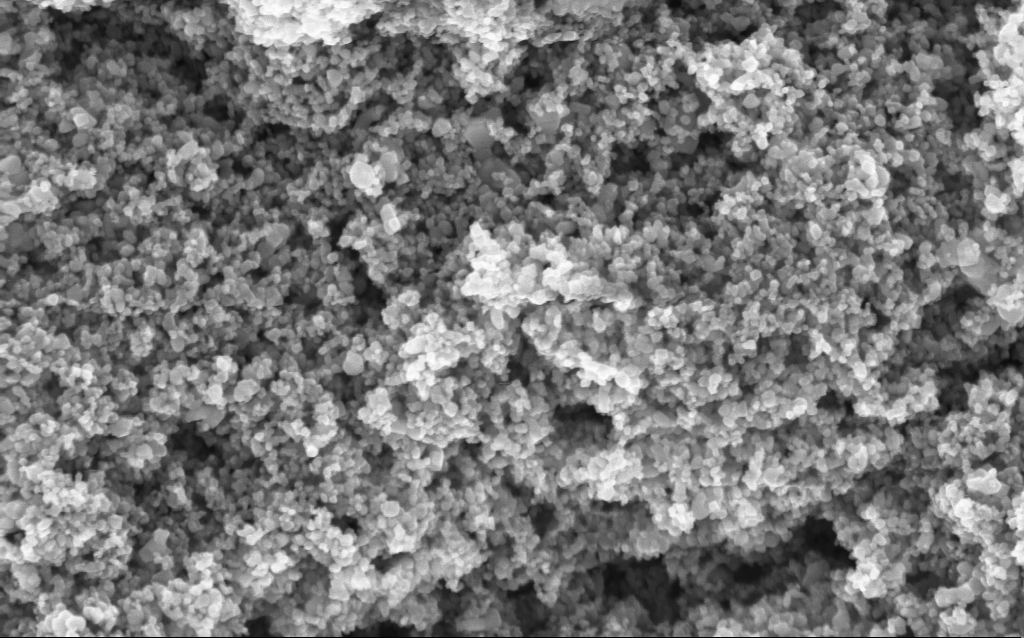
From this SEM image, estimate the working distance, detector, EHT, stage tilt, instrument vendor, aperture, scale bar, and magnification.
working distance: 4.4 mm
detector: InLens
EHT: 5 kV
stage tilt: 0°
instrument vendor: Zeiss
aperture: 30 µm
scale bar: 200 nm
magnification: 114.73 K X